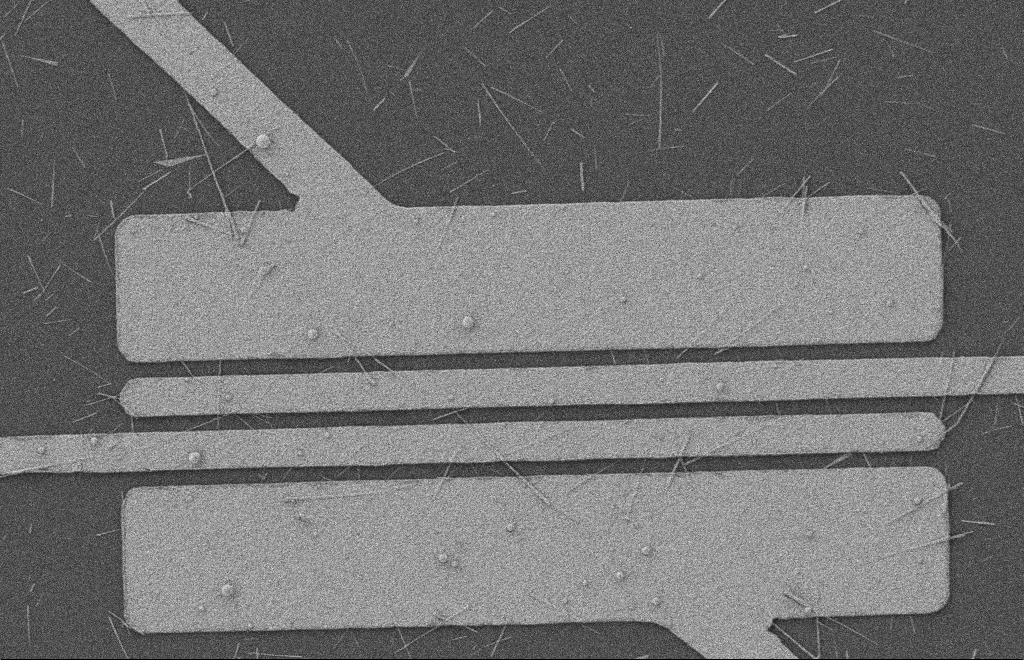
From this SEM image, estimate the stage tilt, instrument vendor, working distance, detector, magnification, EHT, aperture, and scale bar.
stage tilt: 0°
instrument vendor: Zeiss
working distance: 8 mm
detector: SE2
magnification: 4.97 K X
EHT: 2 kV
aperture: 20 µm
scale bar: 2000 nm